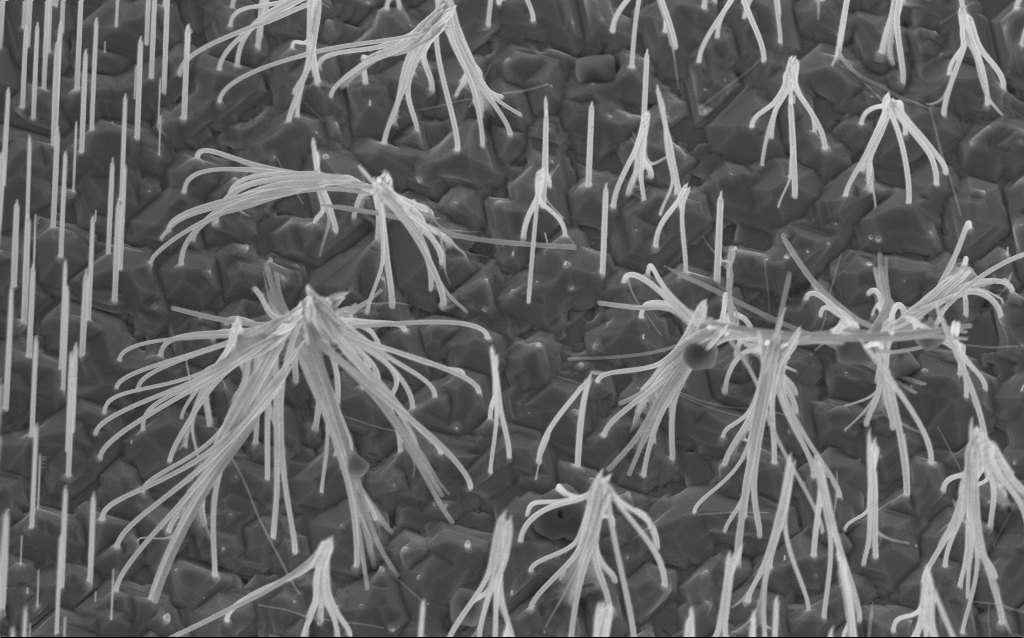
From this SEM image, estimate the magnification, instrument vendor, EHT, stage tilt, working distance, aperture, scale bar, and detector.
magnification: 24.28 K X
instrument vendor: Zeiss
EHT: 5 kV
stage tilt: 20°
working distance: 6.5 mm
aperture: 30 µm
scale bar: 2000 nm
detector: InLens